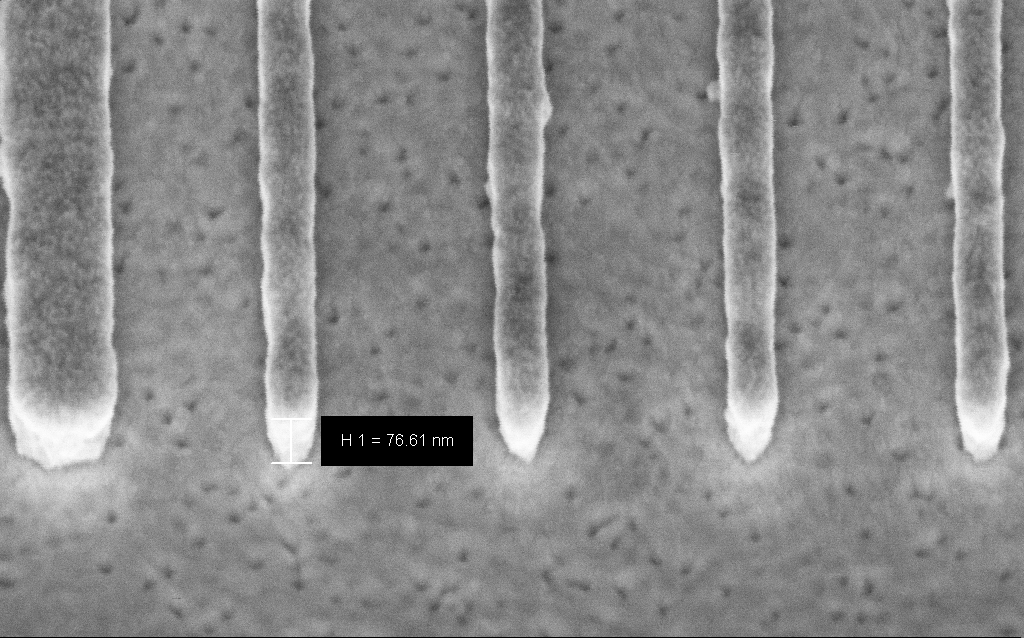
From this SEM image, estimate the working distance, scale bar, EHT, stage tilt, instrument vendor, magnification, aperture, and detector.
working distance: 6.6 mm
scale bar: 200 nm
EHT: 3 kV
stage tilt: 45°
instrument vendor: Zeiss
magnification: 210.88 K X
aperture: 30 µm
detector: InLens